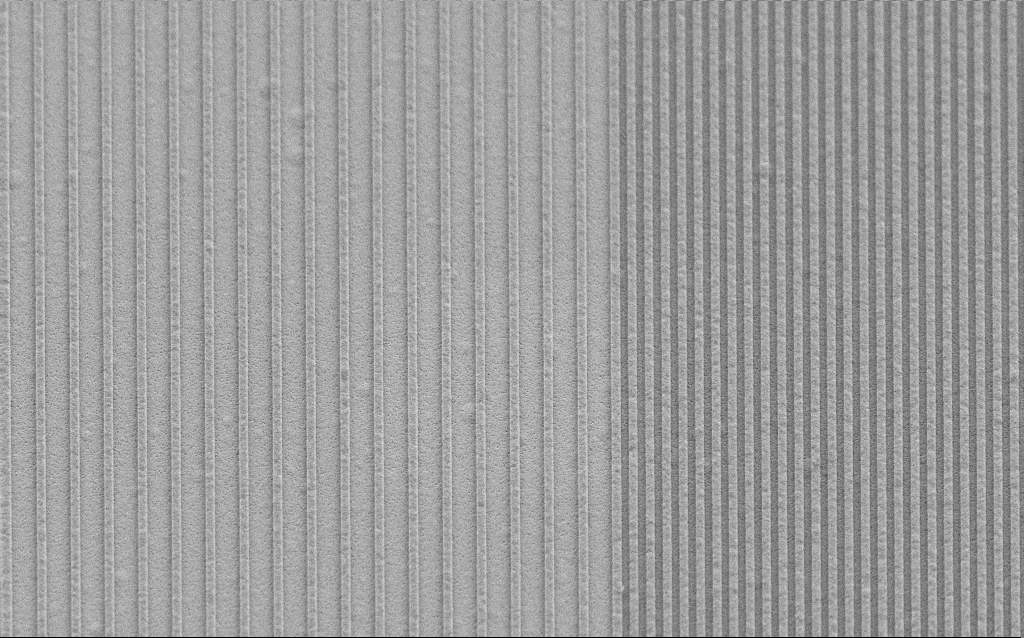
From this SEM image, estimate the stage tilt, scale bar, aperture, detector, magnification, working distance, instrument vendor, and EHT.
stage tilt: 45°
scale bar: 1000 nm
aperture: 30 µm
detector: SE2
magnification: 15.35 K X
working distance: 6.6 mm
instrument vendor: Zeiss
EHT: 3 kV